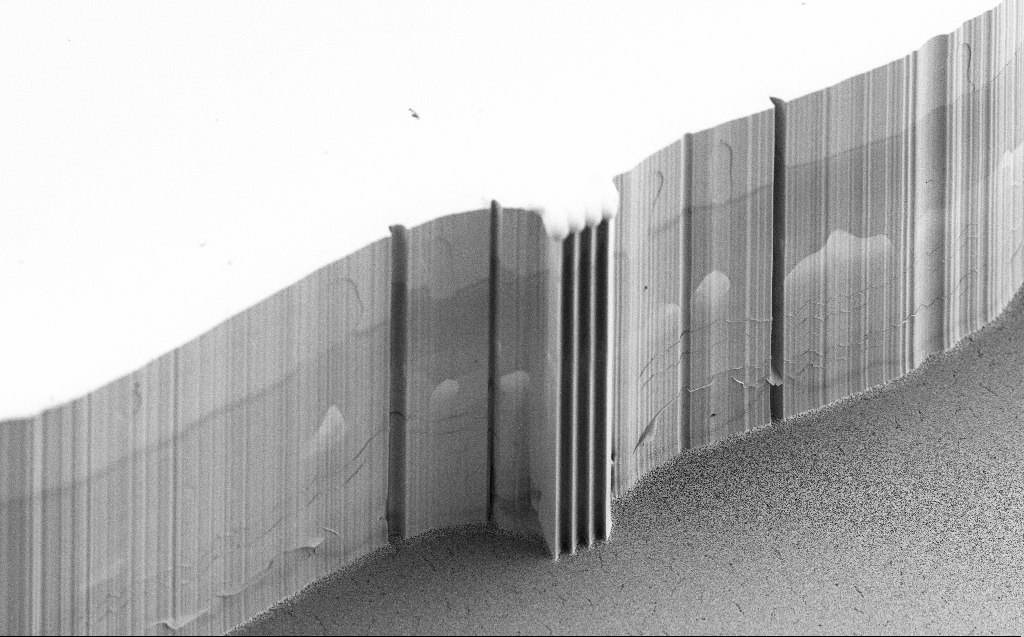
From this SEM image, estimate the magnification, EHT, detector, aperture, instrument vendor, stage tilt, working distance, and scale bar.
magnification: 0.578 K X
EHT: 5 kV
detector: SE2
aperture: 30 µm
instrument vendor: Zeiss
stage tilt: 45°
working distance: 5 mm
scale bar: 100000 nm